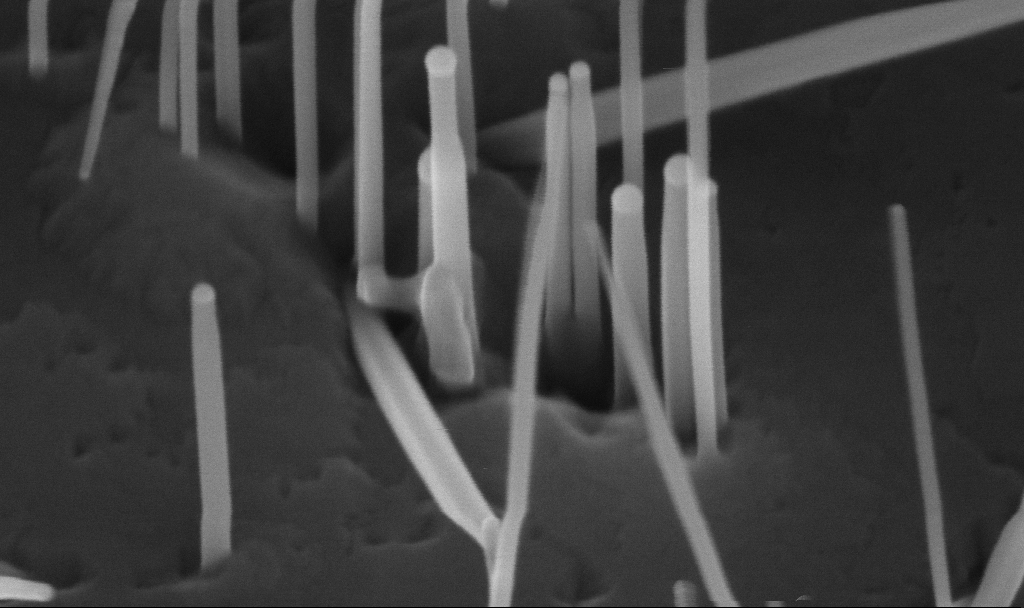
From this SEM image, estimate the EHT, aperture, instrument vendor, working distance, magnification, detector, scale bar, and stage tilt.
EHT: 10 kV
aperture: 30 µm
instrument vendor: Zeiss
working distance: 5.6 mm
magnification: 296.89 K X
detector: InLens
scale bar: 200 nm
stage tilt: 45°